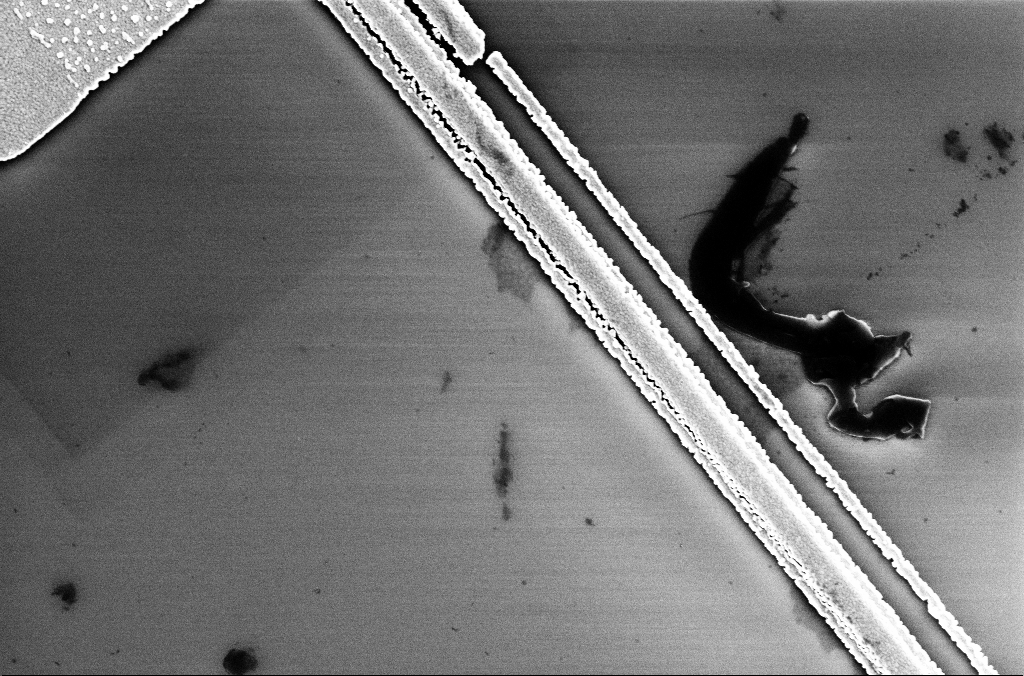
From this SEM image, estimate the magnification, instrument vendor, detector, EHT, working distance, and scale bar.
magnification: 24.92 K X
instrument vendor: Zeiss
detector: InLens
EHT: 5 kV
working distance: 3.4 mm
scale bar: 2000 nm